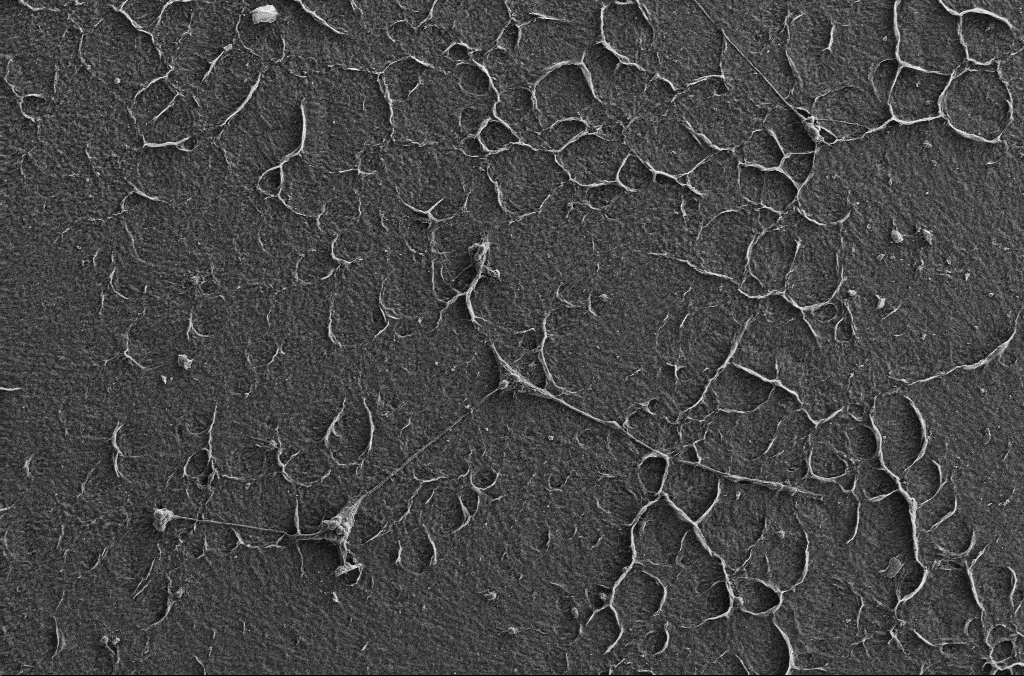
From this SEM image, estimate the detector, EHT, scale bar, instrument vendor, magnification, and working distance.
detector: SE2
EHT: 5 kV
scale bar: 100000 nm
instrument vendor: Zeiss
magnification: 0.5 K X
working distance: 2.9 mm